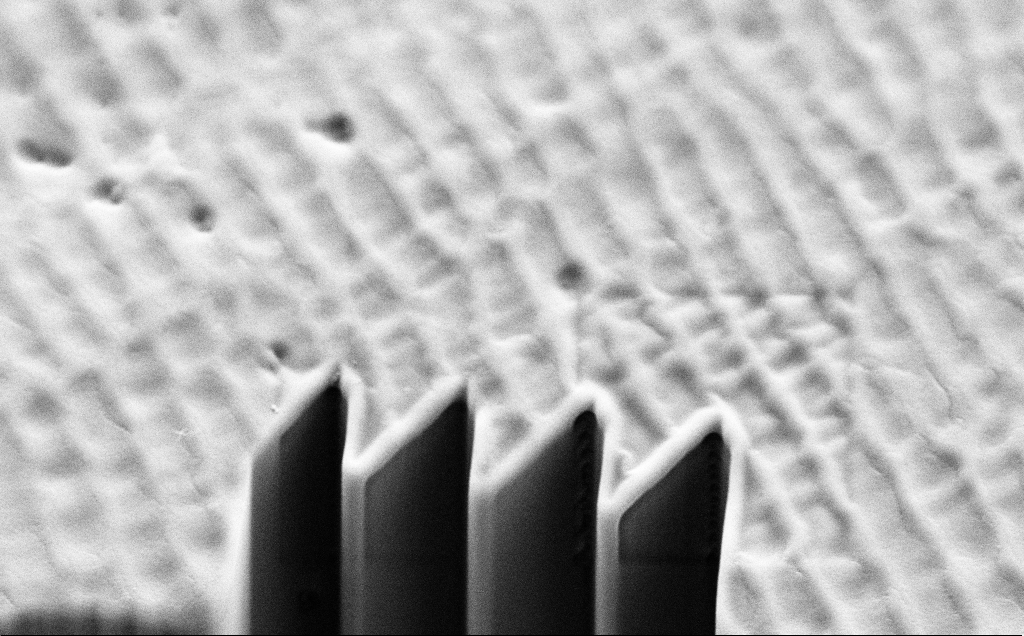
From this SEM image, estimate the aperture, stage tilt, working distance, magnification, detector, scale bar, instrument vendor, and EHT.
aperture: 30 µm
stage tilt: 45°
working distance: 8 mm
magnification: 4.11 K X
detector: SE2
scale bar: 10000 nm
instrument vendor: Zeiss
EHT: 10 kV